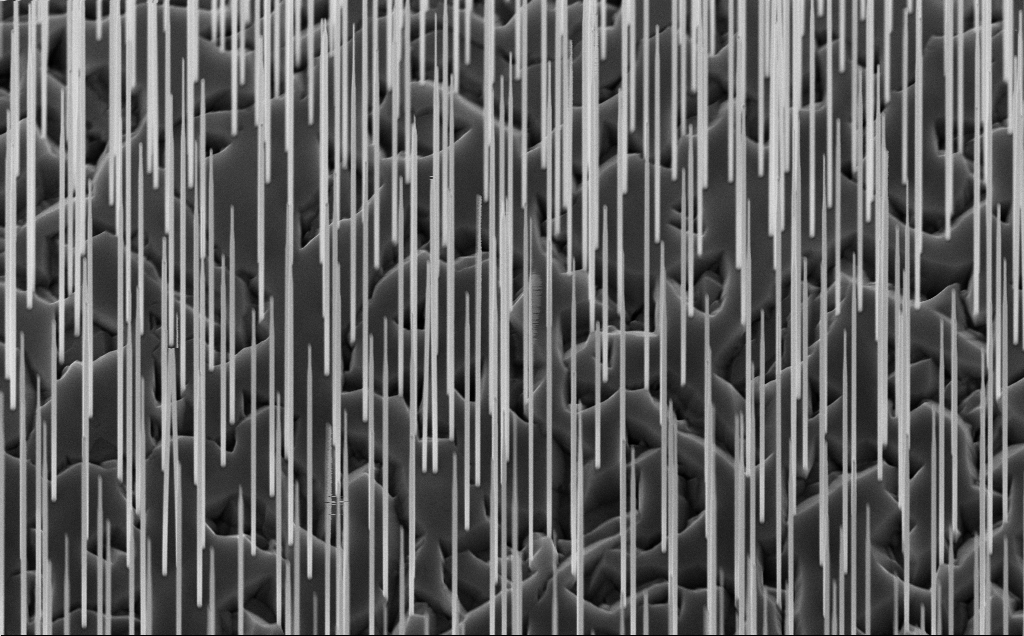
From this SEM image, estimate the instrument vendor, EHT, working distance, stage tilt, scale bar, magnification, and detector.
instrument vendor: Zeiss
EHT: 10 kV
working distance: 7 mm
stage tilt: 45°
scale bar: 2000 nm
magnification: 20.13 K X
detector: InLens